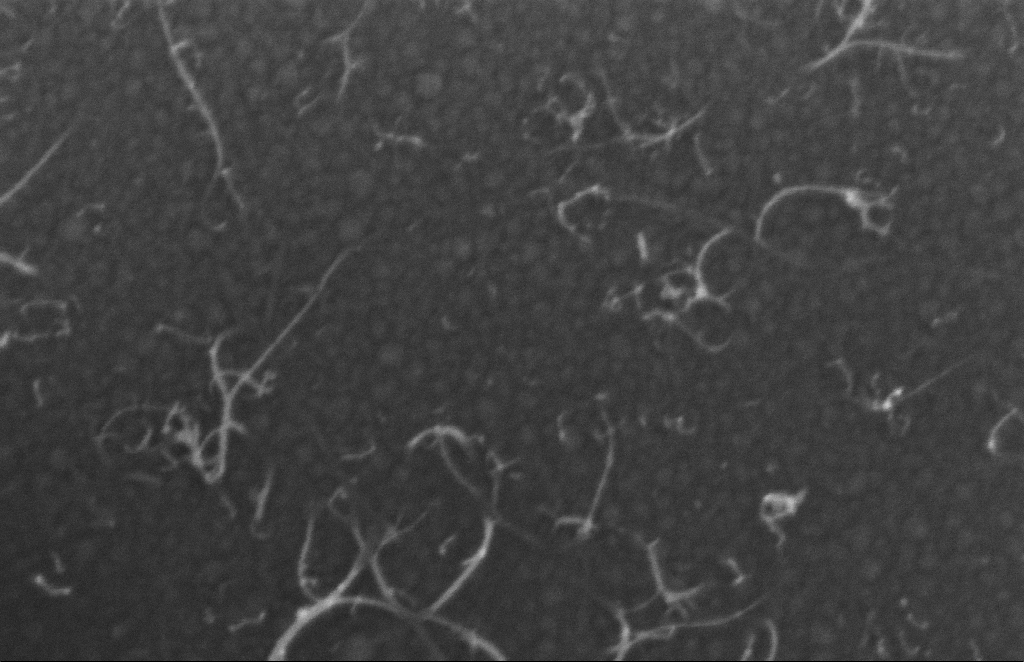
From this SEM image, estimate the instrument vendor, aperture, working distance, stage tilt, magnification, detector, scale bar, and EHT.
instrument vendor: Zeiss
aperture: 20 µm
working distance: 4 mm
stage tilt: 0°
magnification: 415.57 K X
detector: InLens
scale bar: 100 nm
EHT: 5 kV